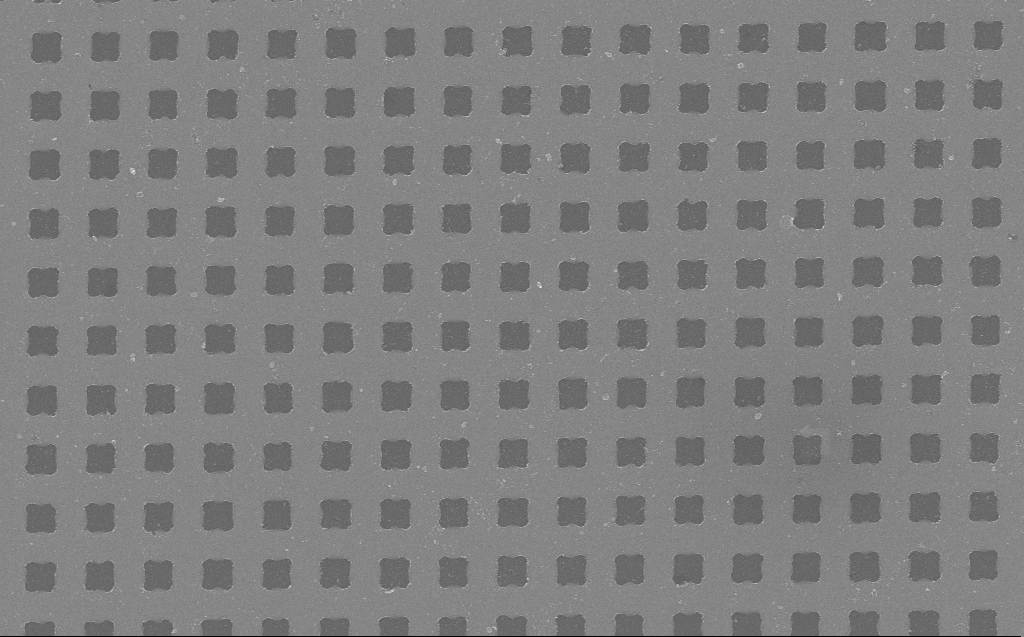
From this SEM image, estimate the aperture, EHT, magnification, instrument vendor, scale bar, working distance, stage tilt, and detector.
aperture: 30 µm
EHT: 10 kV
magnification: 43.68 K X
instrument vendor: Zeiss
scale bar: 1000 nm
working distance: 5 mm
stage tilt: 0°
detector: InLens